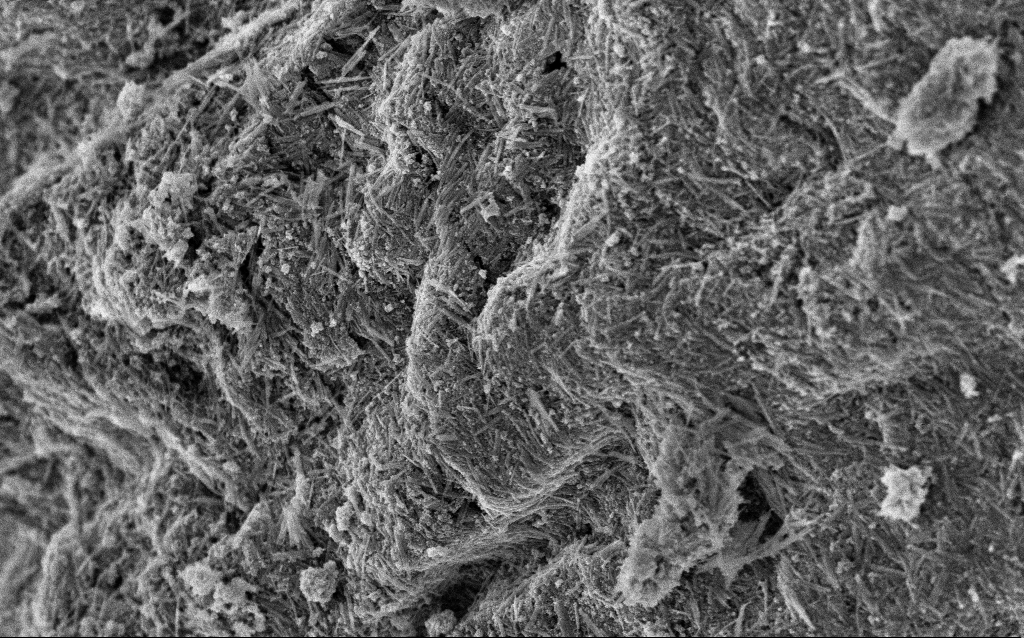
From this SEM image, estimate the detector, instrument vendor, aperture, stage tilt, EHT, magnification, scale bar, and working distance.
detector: InLens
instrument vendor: Zeiss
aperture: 30 µm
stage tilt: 0°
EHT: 3 kV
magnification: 51.3 K X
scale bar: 1000 nm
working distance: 4.9 mm